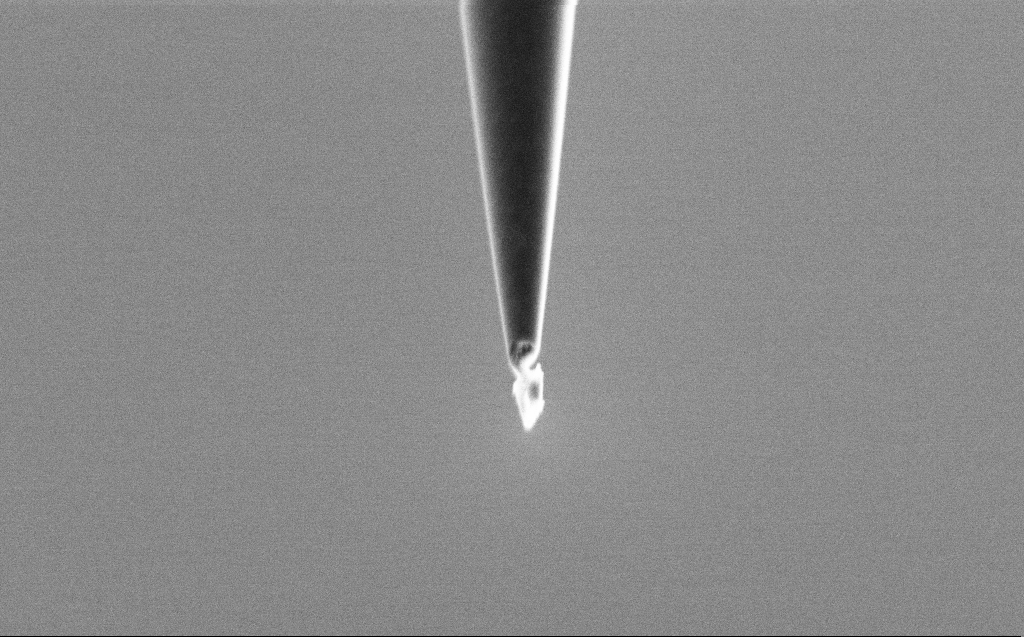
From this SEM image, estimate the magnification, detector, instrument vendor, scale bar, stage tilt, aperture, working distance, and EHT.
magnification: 50 K X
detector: SE2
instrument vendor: Zeiss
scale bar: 1000 nm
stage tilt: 45°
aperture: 30 µm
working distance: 6 mm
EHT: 2 kV